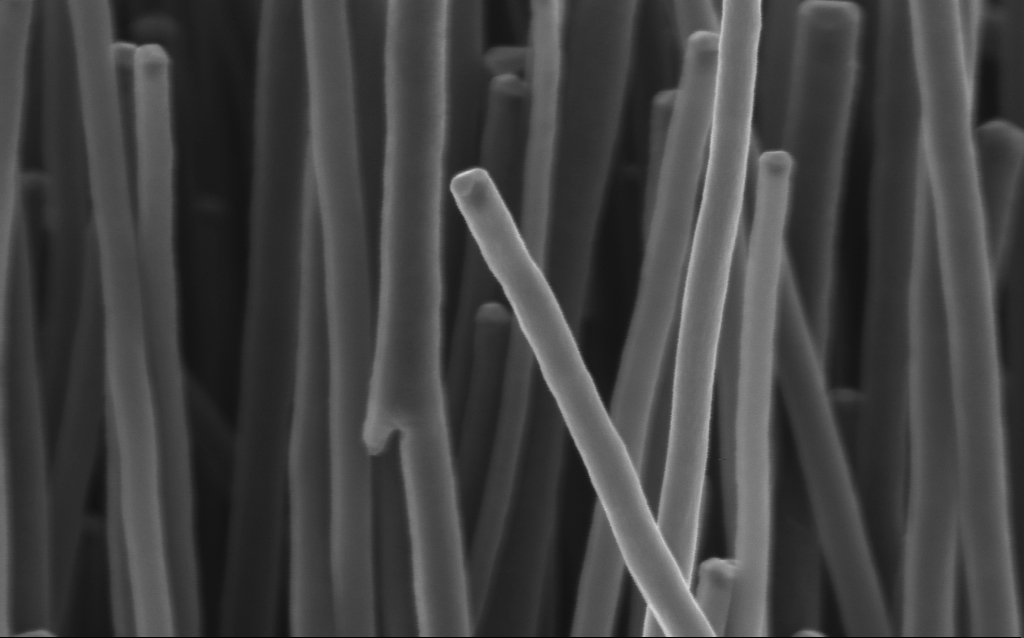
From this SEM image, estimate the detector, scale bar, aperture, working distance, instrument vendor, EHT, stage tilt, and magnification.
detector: InLens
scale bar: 200 nm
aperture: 30 µm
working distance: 3 mm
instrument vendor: Zeiss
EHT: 3 kV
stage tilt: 0°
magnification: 99.8 K X